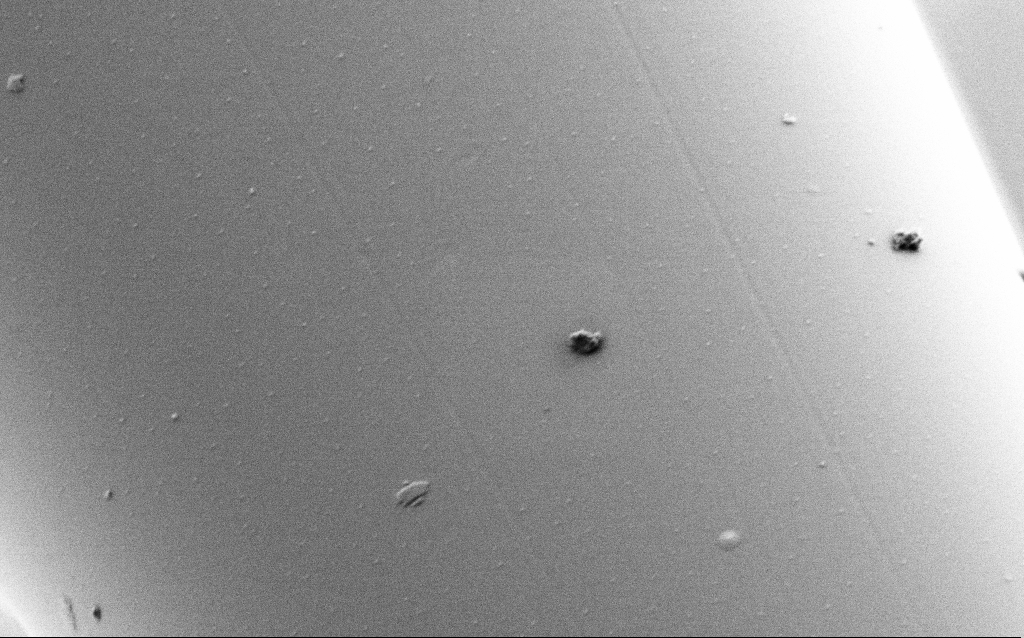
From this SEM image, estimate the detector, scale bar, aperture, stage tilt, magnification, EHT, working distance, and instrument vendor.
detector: SE2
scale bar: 1000 nm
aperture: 30 µm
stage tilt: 45°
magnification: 40 K X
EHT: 1 kV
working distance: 7.4 mm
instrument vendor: Zeiss